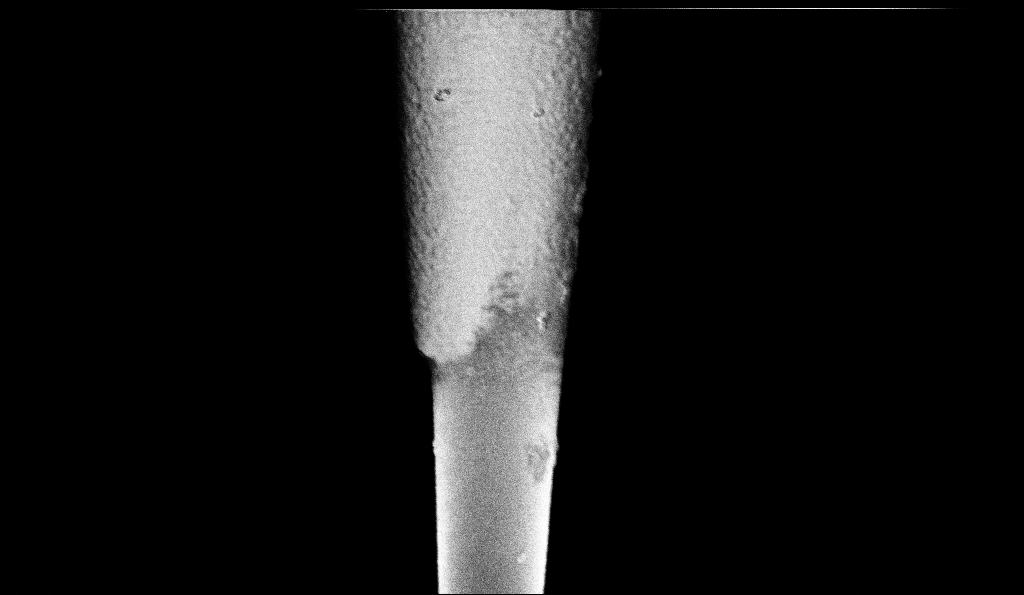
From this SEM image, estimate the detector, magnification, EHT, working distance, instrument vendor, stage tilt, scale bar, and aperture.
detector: InLens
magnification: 25 K X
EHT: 1 kV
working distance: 6.5 mm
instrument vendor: Zeiss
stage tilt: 0°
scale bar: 2000 nm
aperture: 30 µm